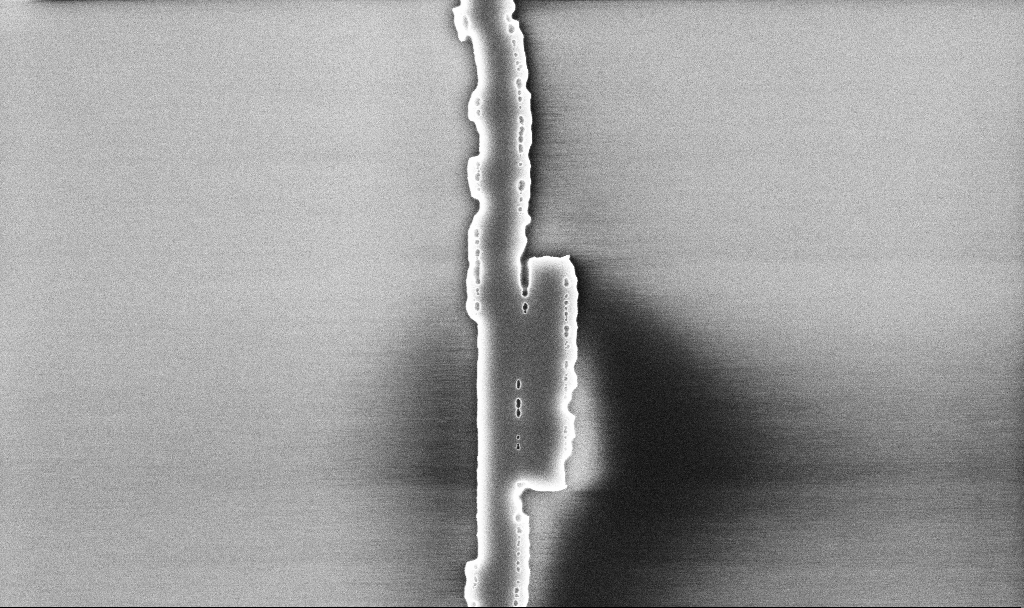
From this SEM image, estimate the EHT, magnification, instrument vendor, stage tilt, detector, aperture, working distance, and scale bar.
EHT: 5 kV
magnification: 28.72 K X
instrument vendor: Zeiss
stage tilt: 0°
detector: InLens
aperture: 30 µm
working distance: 10.1 mm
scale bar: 2000 nm